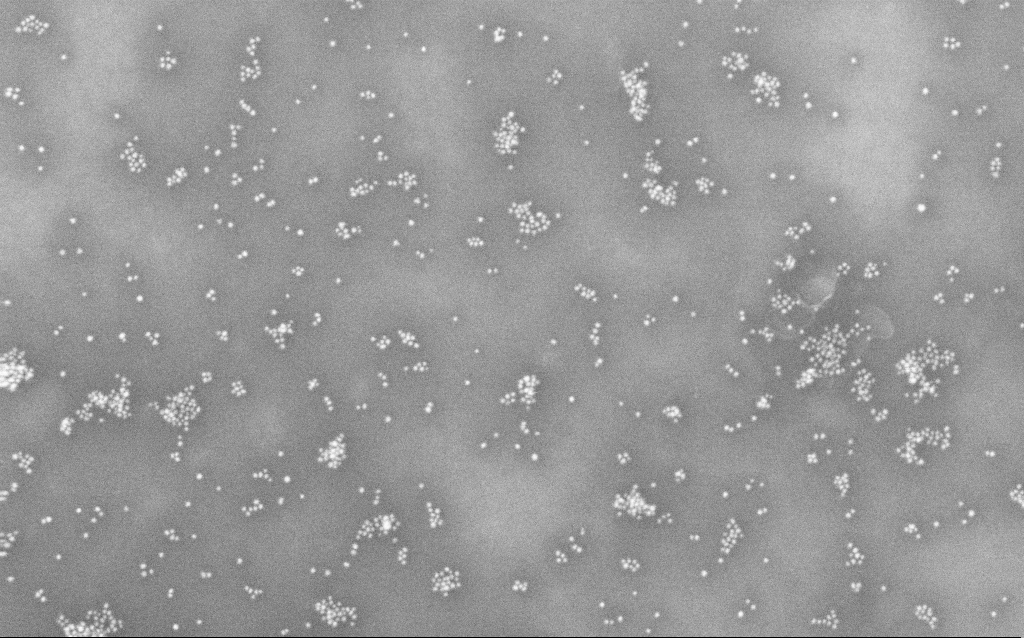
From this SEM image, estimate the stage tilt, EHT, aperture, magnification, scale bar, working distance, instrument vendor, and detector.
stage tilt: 0°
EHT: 10 kV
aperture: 30 µm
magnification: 100 K X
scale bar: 200 nm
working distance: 1.8 mm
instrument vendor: Zeiss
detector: InLens